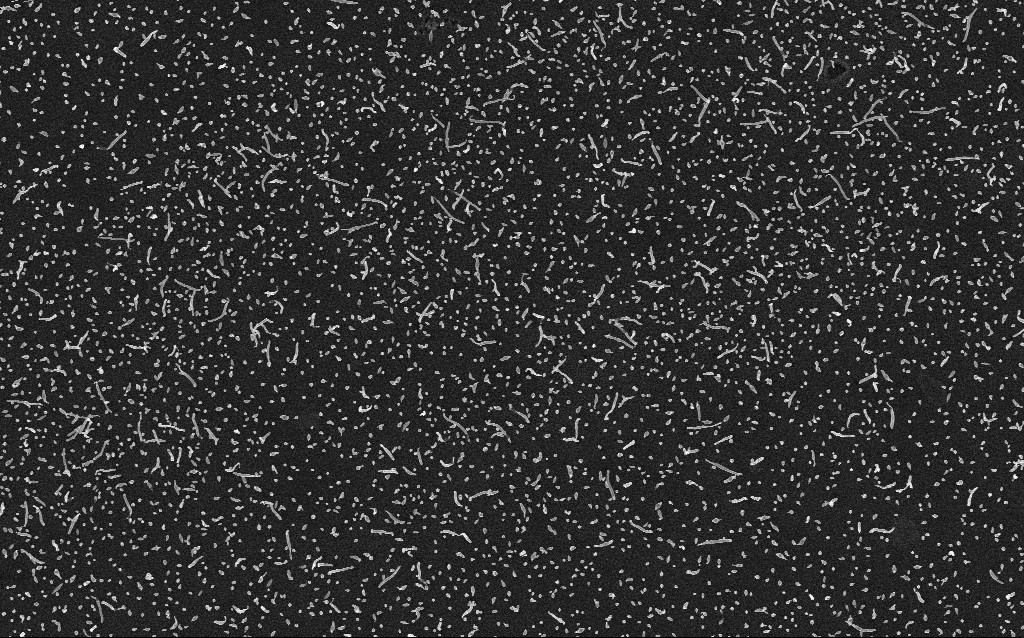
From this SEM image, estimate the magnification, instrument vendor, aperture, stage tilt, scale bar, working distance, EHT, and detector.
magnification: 10 K X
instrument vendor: Zeiss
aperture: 30 µm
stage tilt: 0°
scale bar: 2000 nm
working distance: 2.1 mm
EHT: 5 kV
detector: InLens